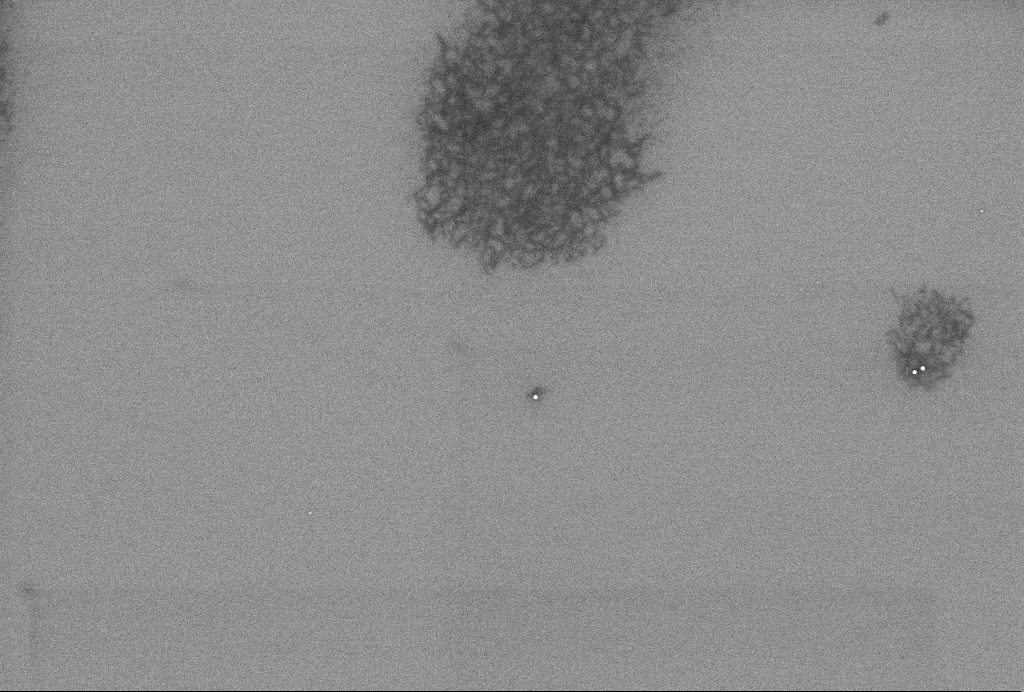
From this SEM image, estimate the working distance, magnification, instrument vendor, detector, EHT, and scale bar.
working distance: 3.3 mm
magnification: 54.42 K X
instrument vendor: Zeiss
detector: InLens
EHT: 2 kV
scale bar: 1000 nm